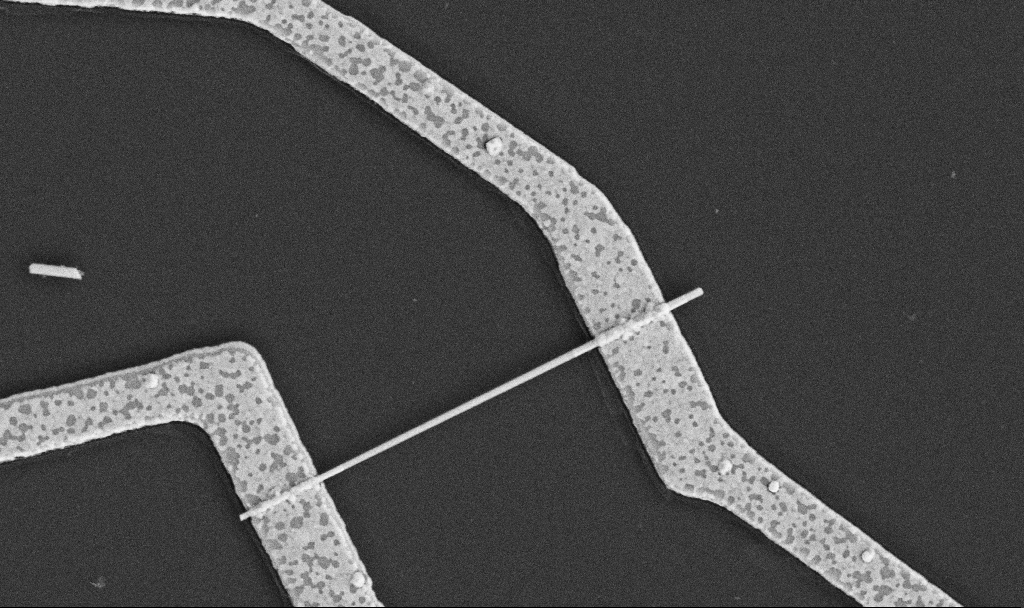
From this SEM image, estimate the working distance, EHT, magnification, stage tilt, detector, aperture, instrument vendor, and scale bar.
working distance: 10.6 mm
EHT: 5 kV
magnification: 30 K X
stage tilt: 0°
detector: SE2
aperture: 30 µm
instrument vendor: Zeiss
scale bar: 1000 nm